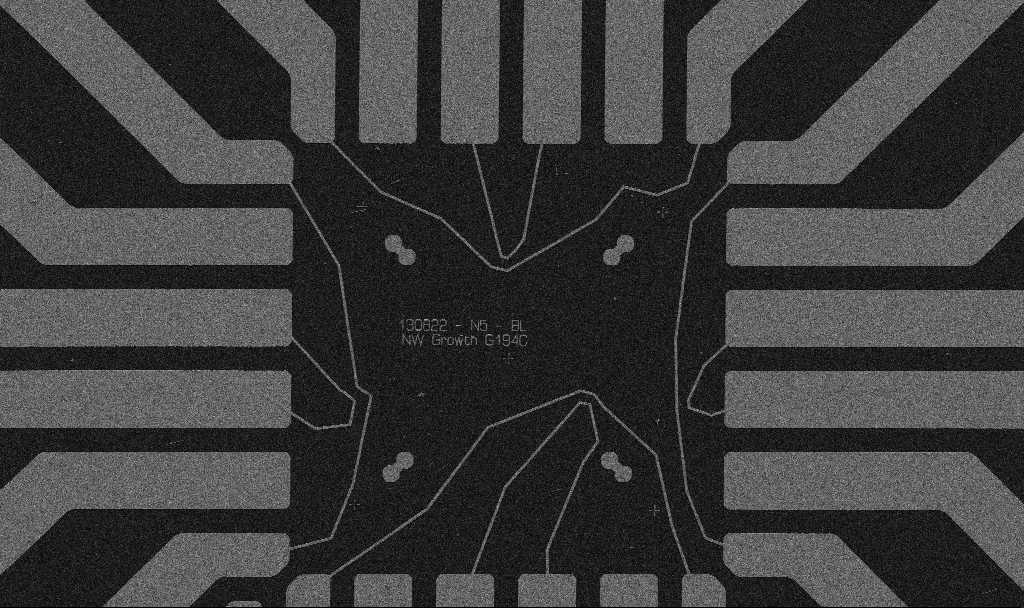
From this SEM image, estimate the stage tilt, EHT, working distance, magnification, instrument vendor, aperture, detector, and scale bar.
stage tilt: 0°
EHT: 5 kV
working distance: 10.7 mm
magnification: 1 K X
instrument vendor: Zeiss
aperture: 30 µm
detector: SE2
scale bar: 20000 nm